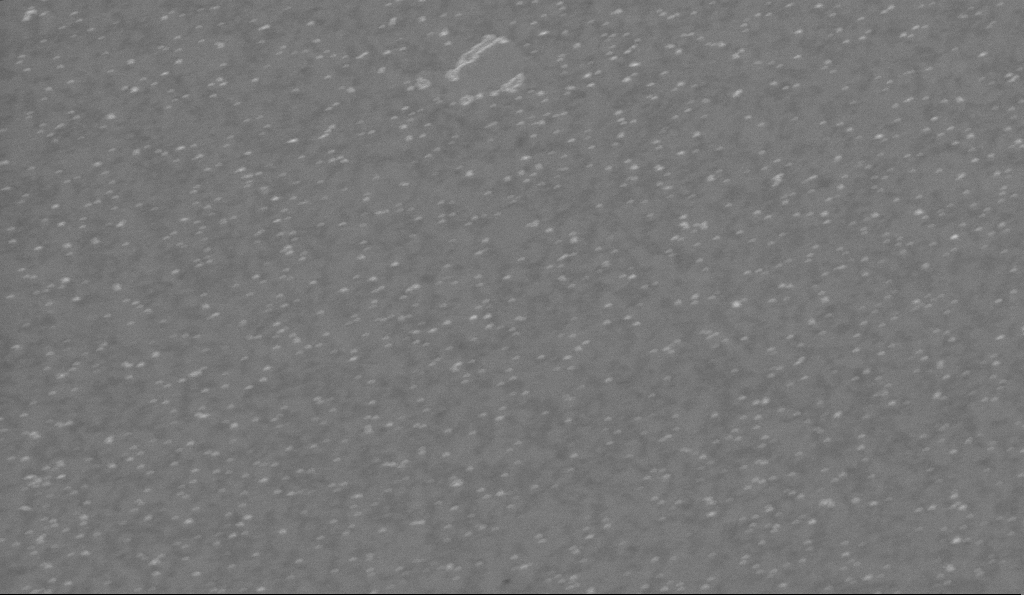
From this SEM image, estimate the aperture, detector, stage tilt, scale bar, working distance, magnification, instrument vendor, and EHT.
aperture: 30 µm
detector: InLens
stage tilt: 0°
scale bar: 1000 nm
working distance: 3.4 mm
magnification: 25 K X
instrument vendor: Zeiss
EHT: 2 kV